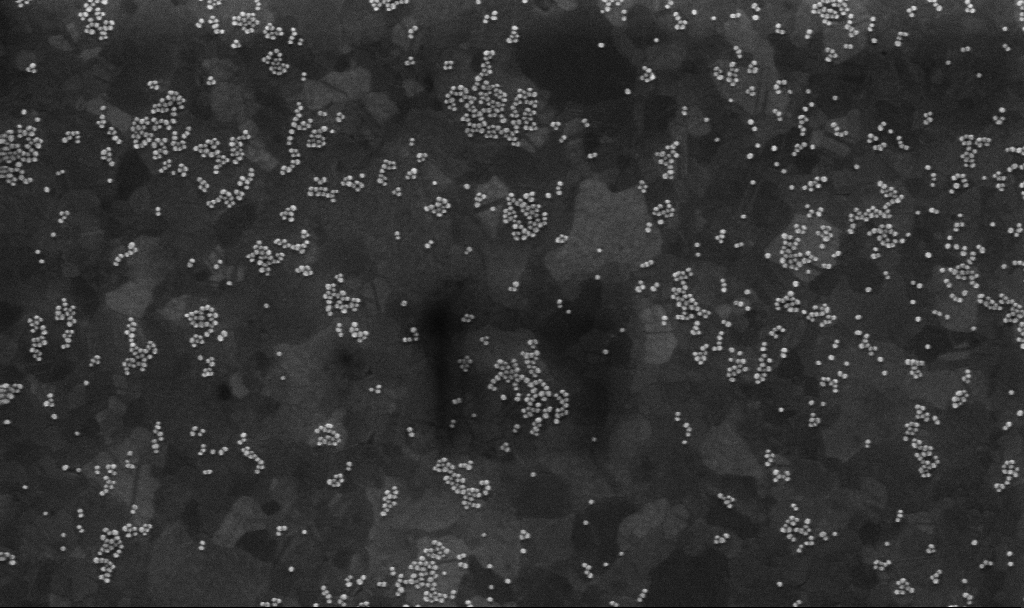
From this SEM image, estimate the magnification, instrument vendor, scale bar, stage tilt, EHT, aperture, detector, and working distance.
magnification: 103.66 K X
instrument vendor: Zeiss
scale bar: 200 nm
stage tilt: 0°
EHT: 10 kV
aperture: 30 µm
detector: InLens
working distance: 3.8 mm